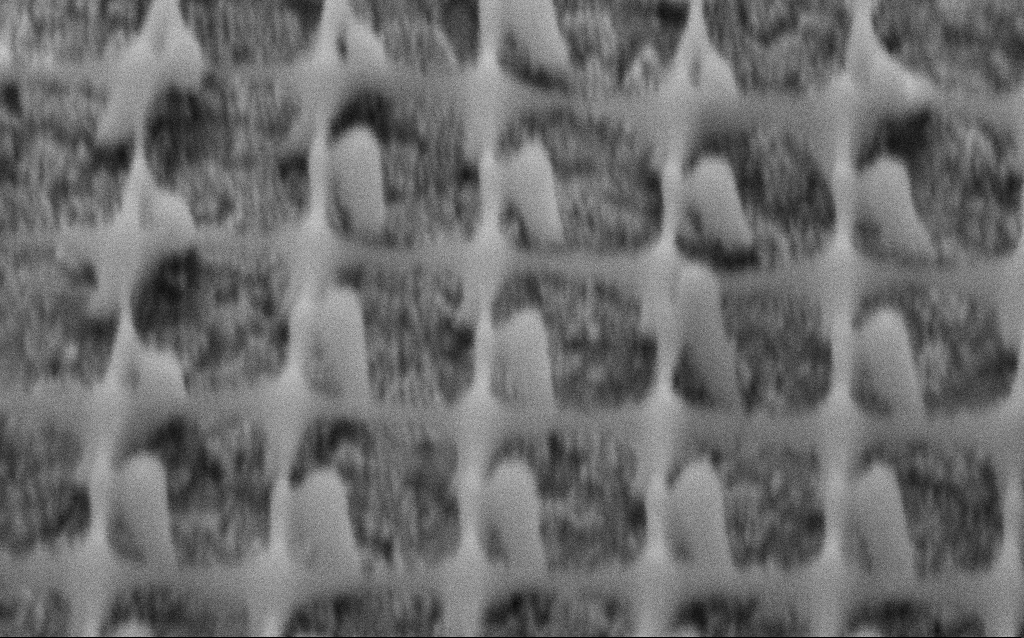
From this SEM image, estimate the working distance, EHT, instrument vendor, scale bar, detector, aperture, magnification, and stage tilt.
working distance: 9.7 mm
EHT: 3 kV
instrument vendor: Zeiss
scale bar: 200 nm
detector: SE2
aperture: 30 µm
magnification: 147.54 K X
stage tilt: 45°